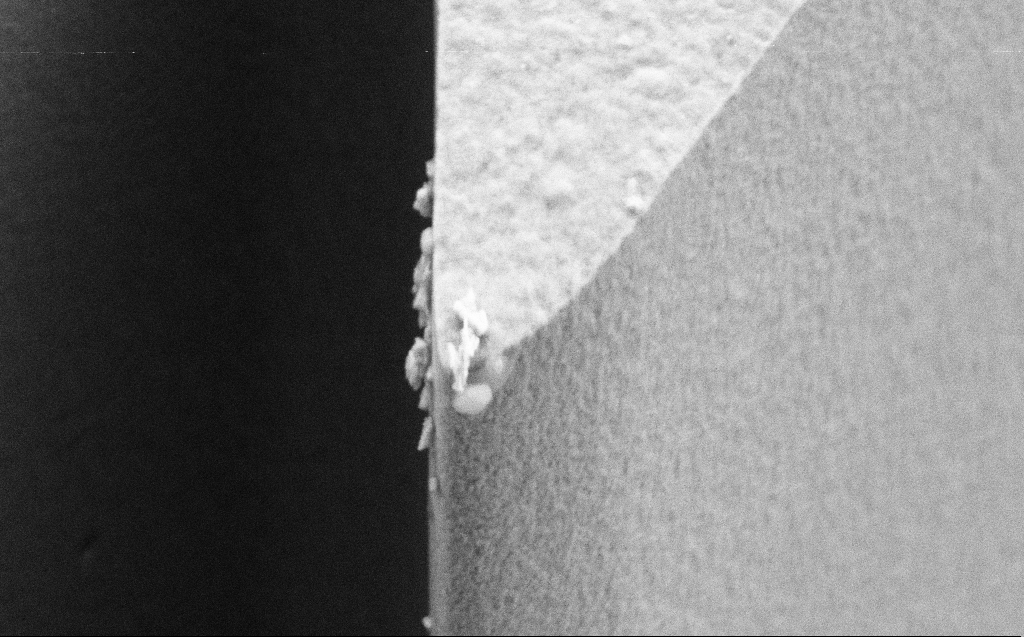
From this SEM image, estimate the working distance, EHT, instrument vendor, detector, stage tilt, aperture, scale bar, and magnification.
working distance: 5 mm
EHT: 5 kV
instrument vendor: Zeiss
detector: SE2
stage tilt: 45°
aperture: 30 µm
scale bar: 1000 nm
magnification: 33.13 K X